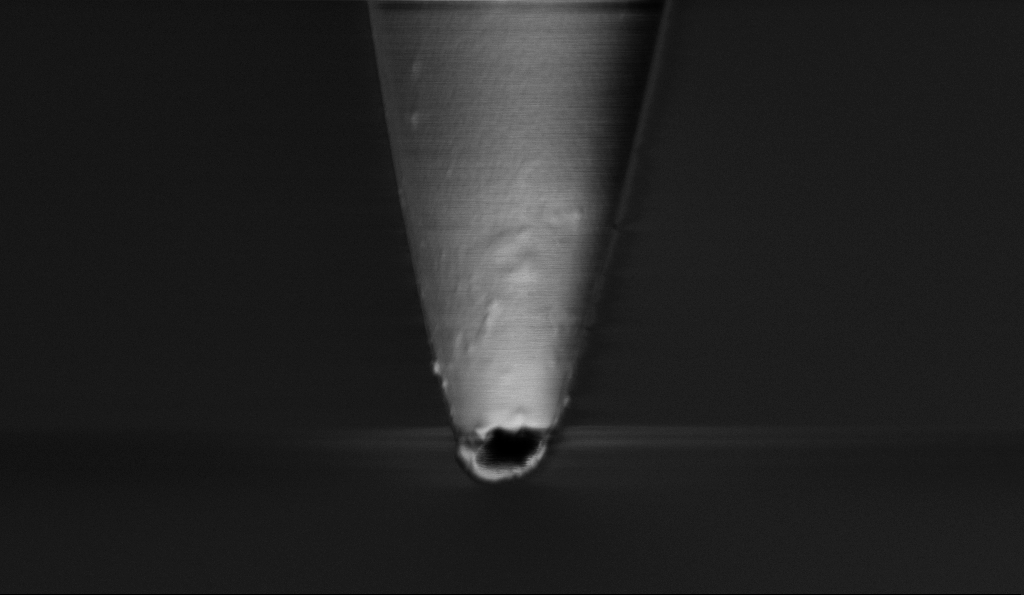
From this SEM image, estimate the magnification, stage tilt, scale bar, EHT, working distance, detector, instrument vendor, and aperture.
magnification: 15 K X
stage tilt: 45°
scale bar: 1000 nm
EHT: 1 kV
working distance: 6.8 mm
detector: InLens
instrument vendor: Zeiss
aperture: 30 µm